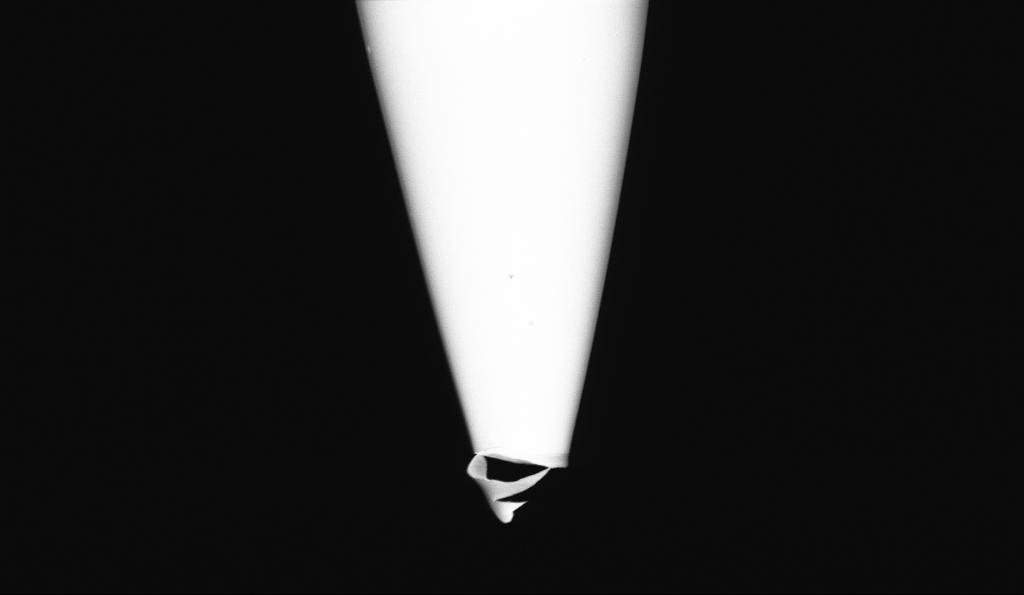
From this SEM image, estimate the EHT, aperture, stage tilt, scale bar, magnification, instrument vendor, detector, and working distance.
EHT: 1 kV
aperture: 30 µm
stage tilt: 0°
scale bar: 1000 nm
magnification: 15 K X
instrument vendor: Zeiss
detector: InLens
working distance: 5.6 mm